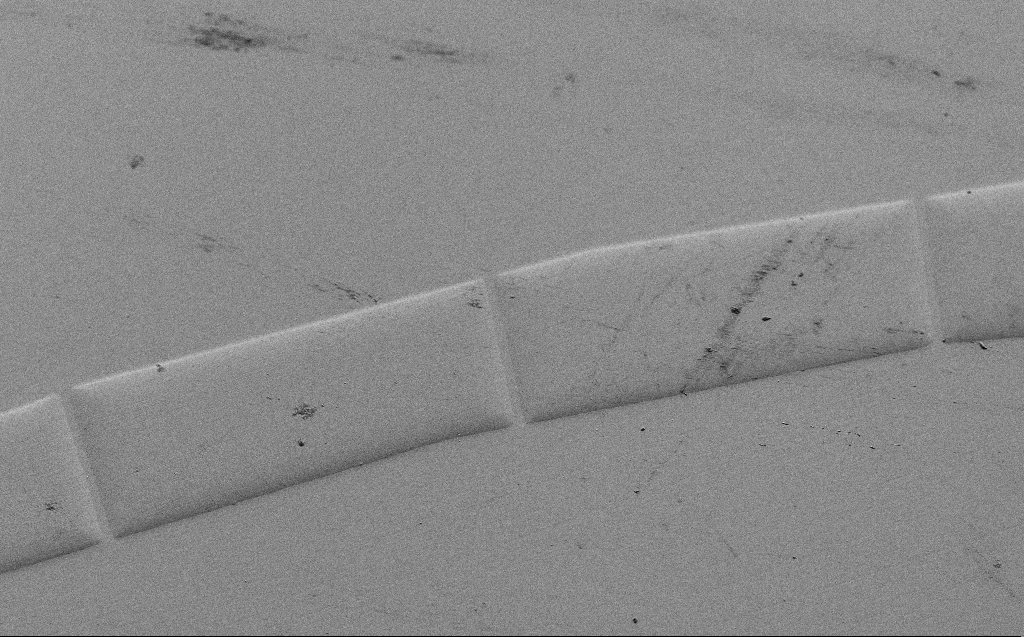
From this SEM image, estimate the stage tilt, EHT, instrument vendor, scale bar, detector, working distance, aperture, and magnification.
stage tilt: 45°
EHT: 5 kV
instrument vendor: Zeiss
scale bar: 100000 nm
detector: SE2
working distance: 8 mm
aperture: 30 µm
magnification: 0.443 K X